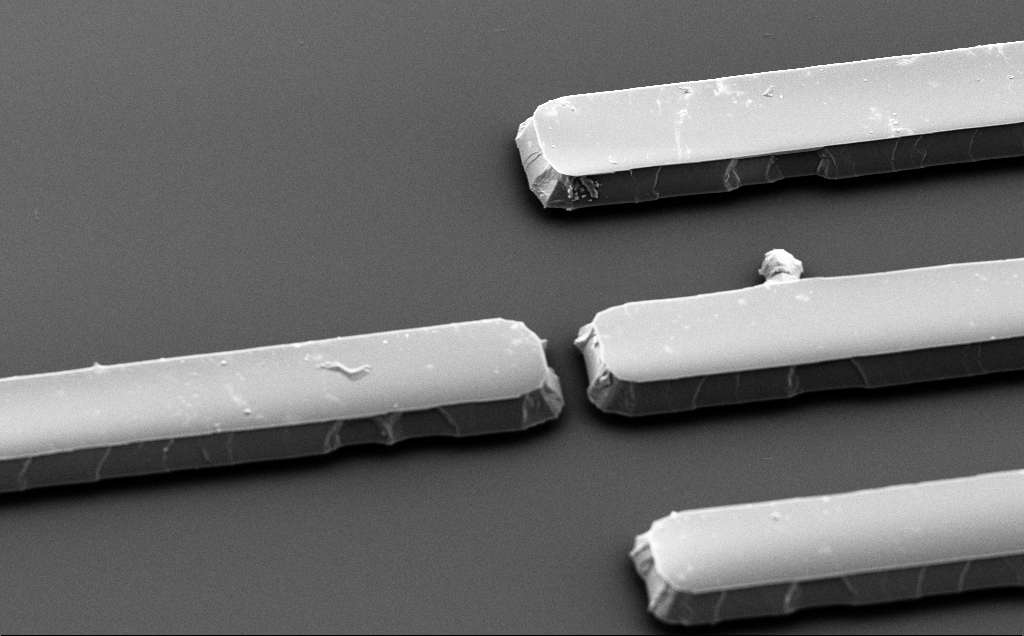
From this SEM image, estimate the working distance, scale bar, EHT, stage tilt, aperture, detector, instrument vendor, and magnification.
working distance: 10 mm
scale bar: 10000 nm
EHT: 5 kV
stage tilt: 50°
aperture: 30 µm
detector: SE2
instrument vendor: Zeiss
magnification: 3.1 K X